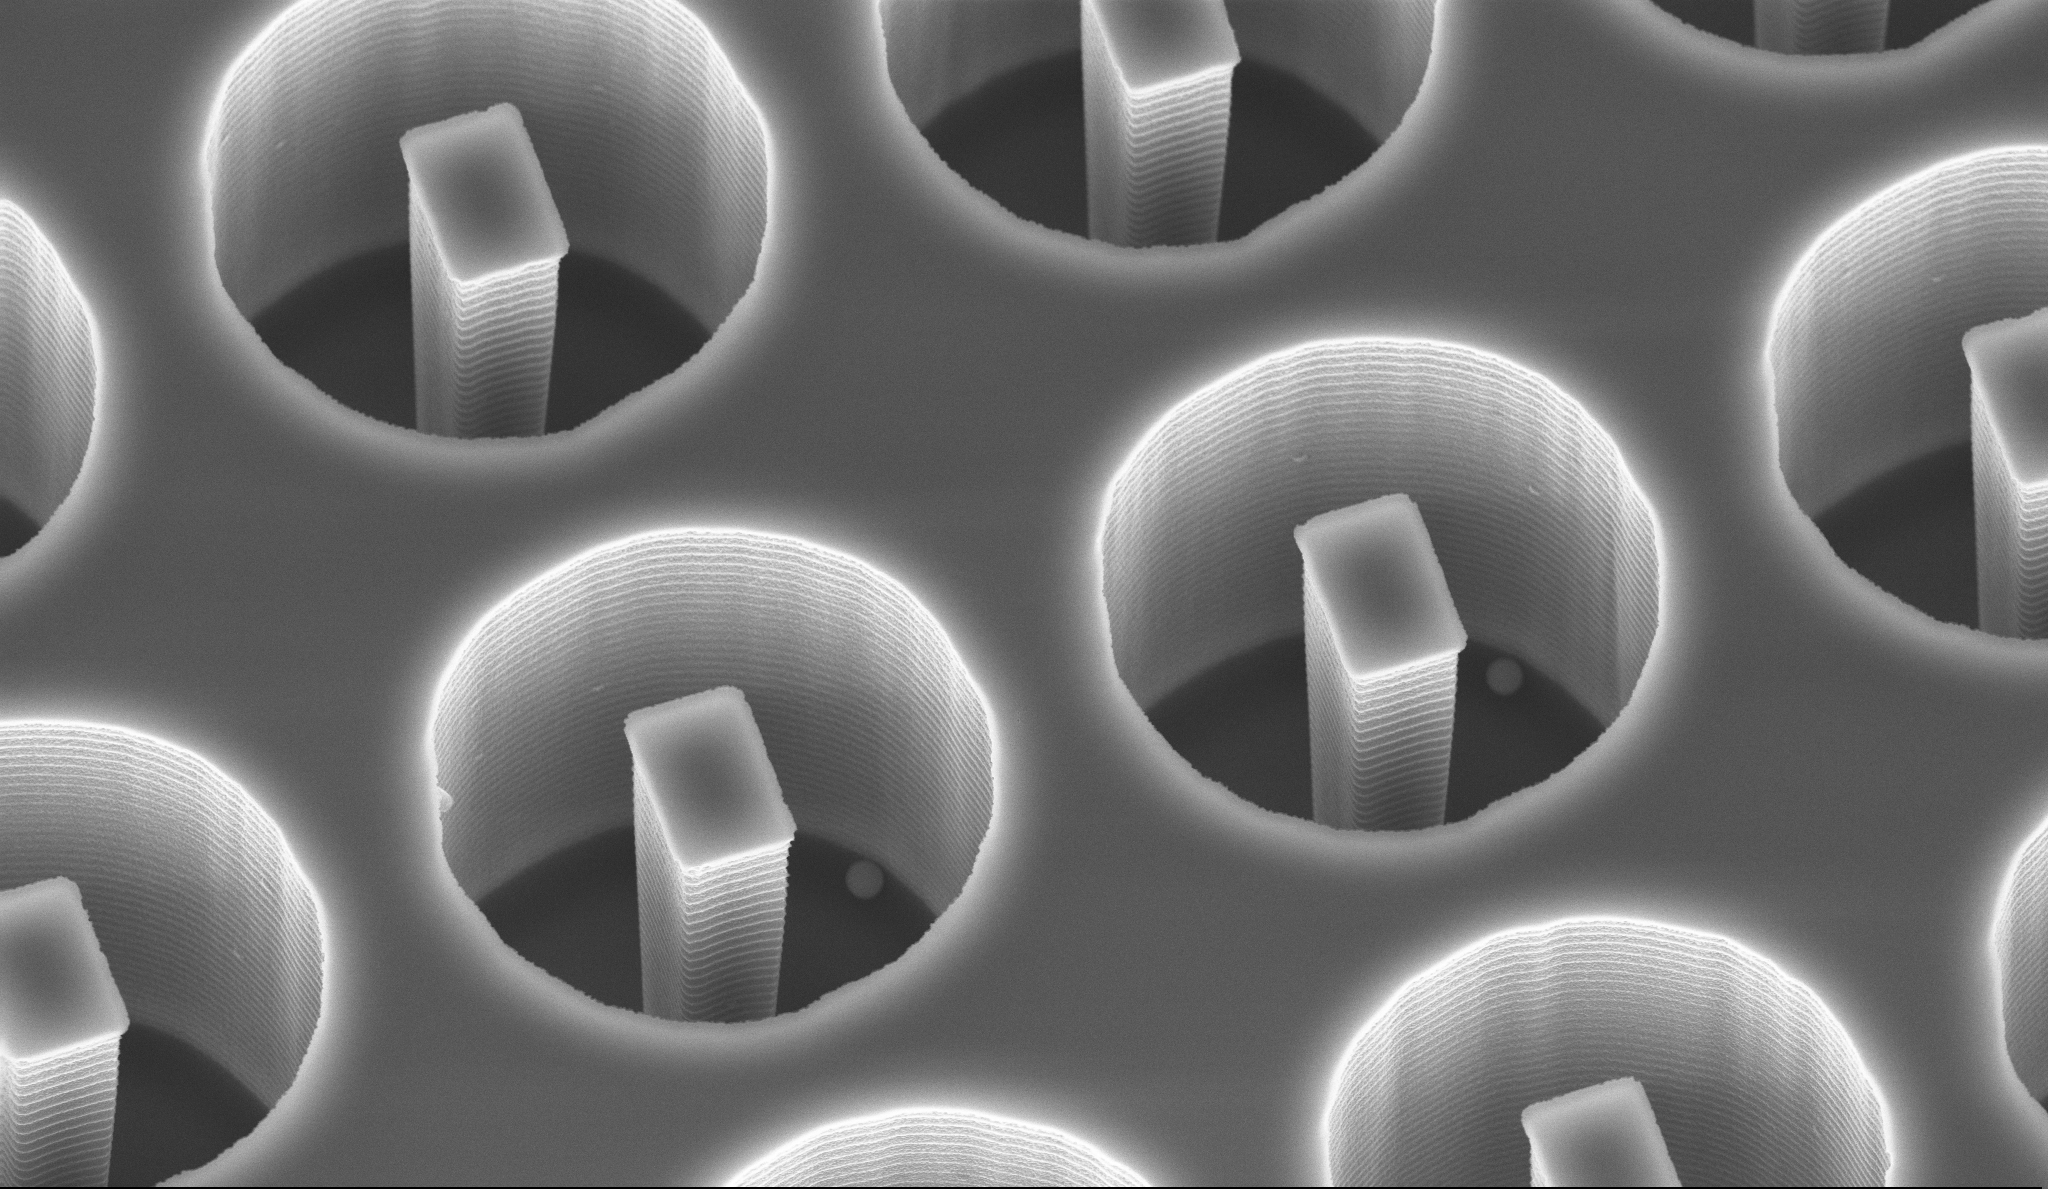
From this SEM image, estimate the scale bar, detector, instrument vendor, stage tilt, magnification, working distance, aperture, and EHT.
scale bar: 2000 nm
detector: InLens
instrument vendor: Zeiss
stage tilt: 30°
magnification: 11.51 K X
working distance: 14.1 mm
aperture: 30 µm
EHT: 10 kV